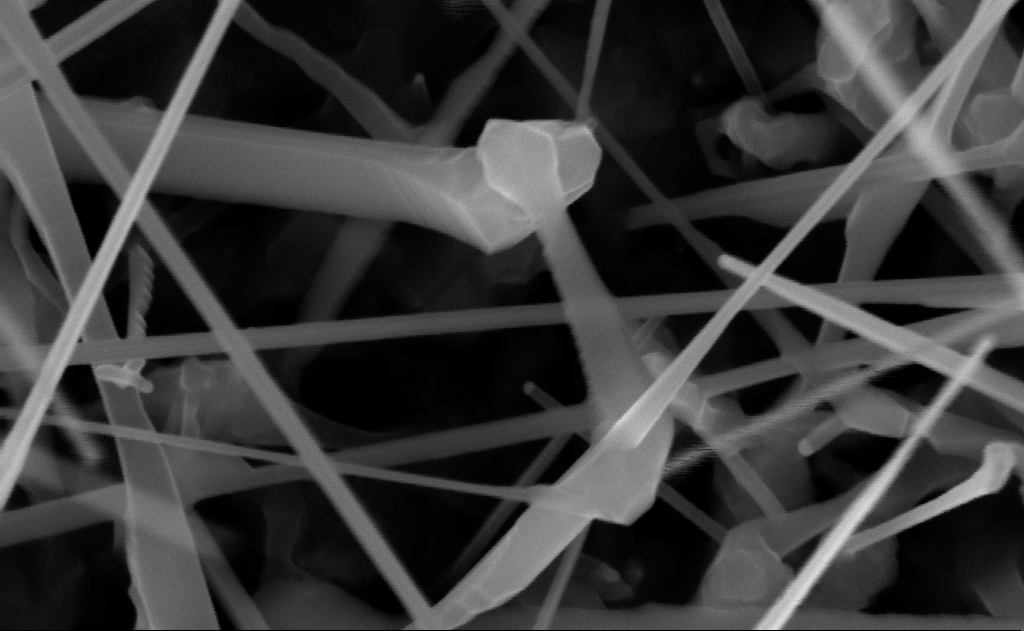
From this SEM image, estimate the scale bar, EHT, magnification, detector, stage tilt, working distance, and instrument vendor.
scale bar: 200 nm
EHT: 10 kV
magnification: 200 K X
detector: InLens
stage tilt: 0°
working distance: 10 mm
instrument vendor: Zeiss